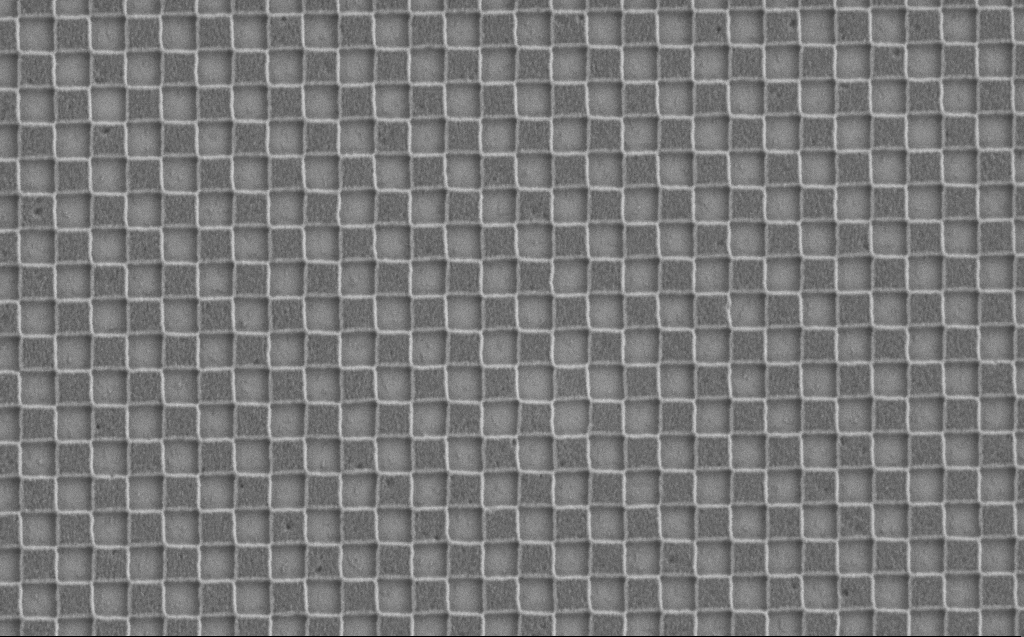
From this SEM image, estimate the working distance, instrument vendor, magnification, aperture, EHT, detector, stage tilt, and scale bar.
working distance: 5 mm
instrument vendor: Zeiss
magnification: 26 K X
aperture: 30 µm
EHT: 1.2 kV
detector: SE2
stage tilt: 0°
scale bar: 1000 nm